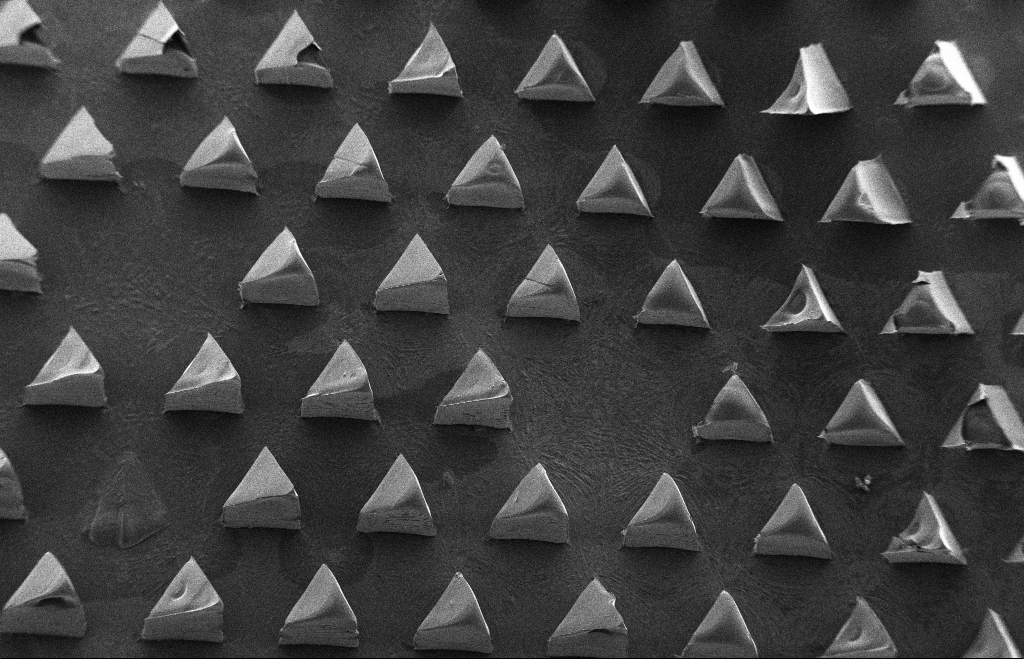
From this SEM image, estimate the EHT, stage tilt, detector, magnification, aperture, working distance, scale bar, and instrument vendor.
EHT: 5 kV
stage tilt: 14.7°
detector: SE2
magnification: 0.104 K X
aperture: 30 µm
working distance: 13 mm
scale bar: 100000 nm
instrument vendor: Zeiss